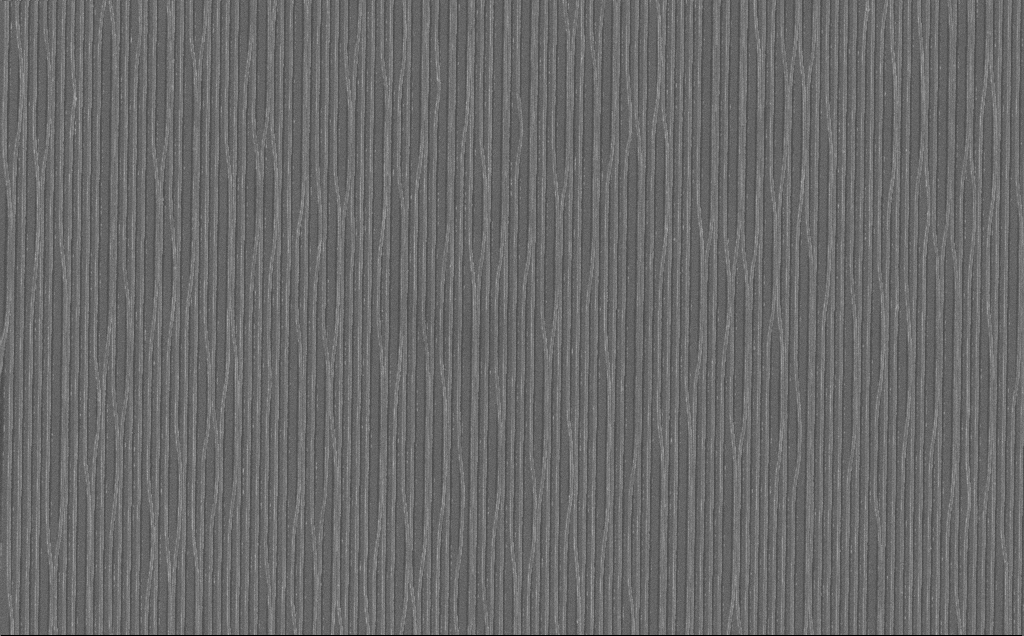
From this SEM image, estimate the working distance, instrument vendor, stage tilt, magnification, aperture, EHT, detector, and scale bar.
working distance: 7 mm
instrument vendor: Zeiss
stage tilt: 0°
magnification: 9.59 K X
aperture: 30 µm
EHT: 10 kV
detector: InLens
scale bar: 2000 nm